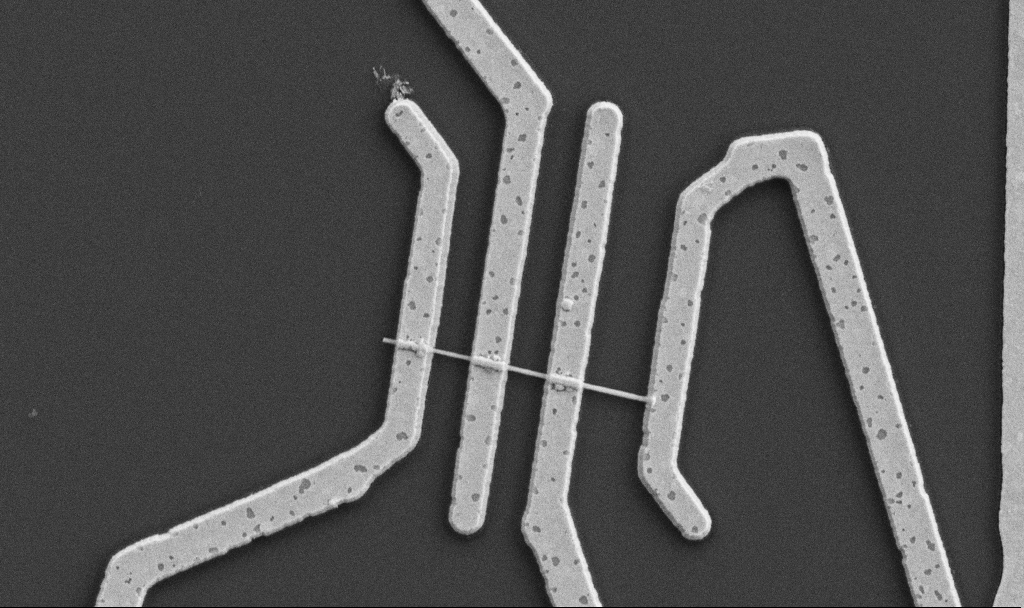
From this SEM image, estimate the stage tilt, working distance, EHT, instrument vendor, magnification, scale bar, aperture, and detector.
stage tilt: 0°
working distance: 10.7 mm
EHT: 5 kV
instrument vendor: Zeiss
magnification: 20 K X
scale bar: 1000 nm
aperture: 30 µm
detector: SE2